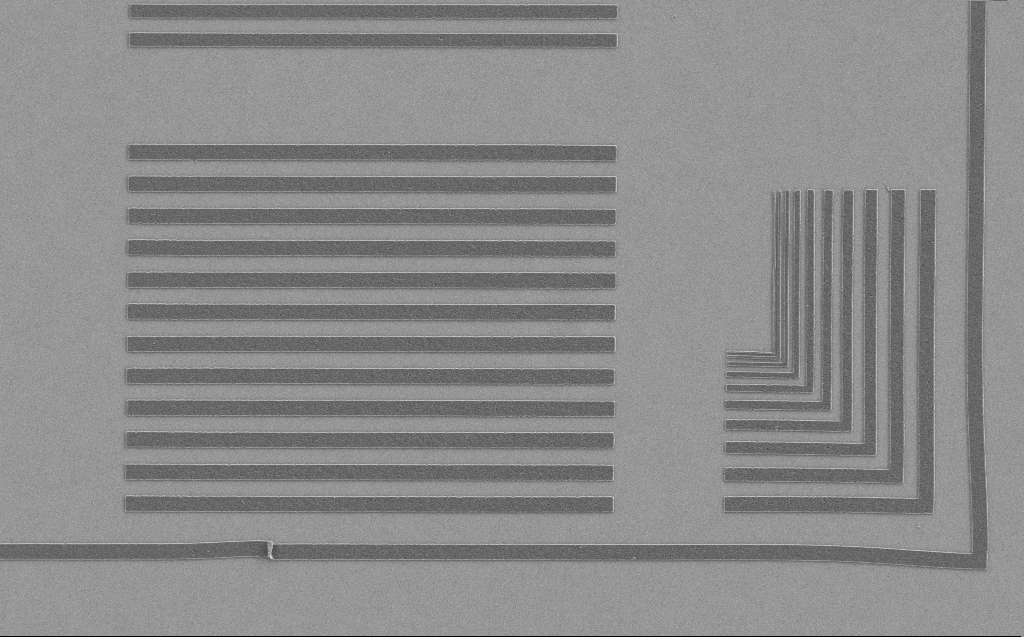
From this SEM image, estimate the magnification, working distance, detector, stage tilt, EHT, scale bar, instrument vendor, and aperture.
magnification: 5.93 K X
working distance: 5 mm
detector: SE2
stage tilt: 0°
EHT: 1.2 kV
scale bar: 10000 nm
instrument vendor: Zeiss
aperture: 30 µm